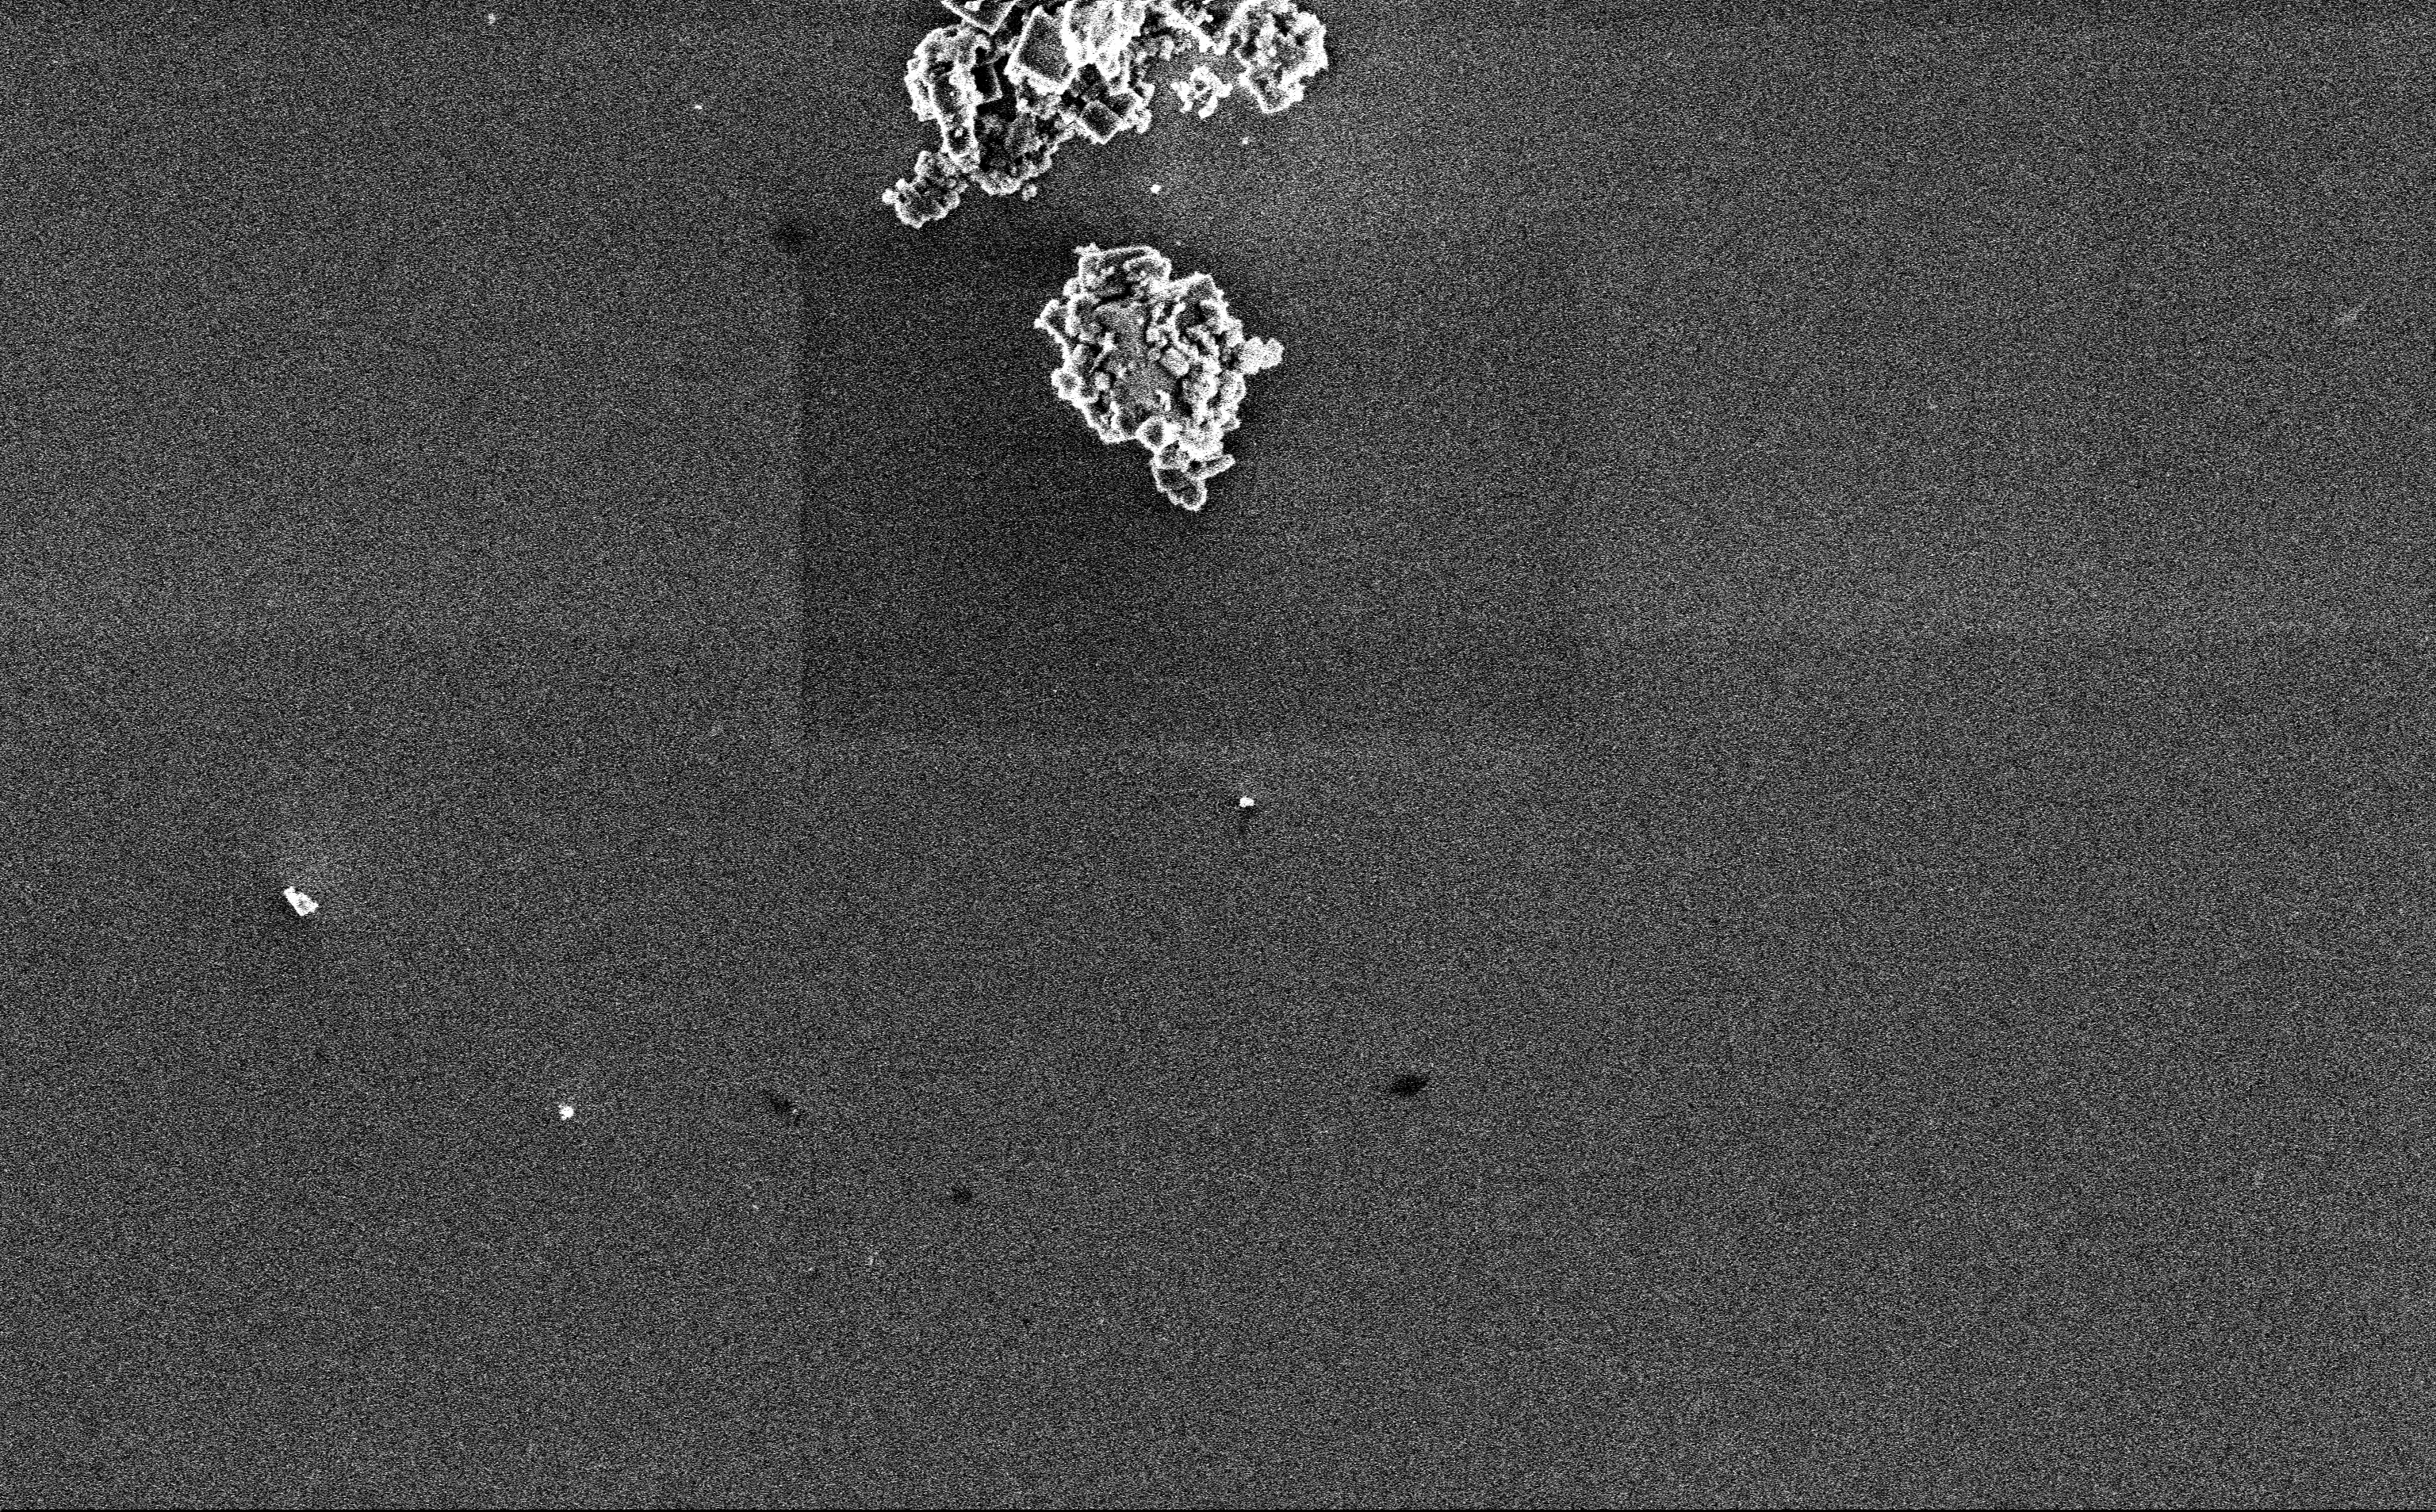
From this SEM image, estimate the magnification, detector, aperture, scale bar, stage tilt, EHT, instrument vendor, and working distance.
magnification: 12.85 K X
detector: InLens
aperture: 30 µm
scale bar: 2000 nm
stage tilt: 0°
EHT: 3 kV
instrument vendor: Zeiss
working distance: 3 mm